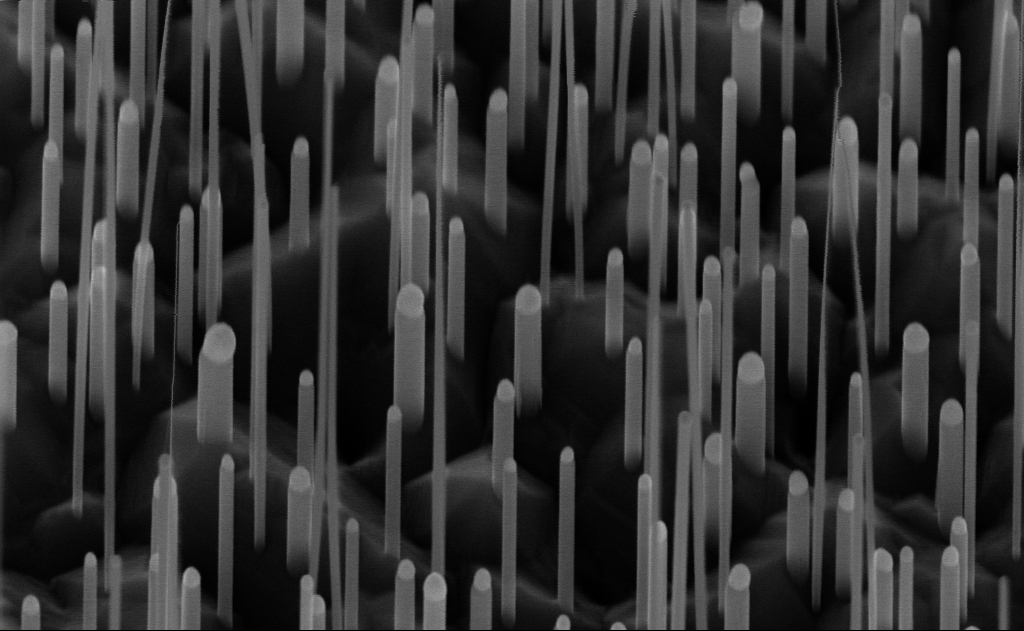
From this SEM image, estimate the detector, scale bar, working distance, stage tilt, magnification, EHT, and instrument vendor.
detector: InLens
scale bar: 200 nm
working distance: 6 mm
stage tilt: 45°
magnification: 100 K X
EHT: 10 kV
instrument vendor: Zeiss